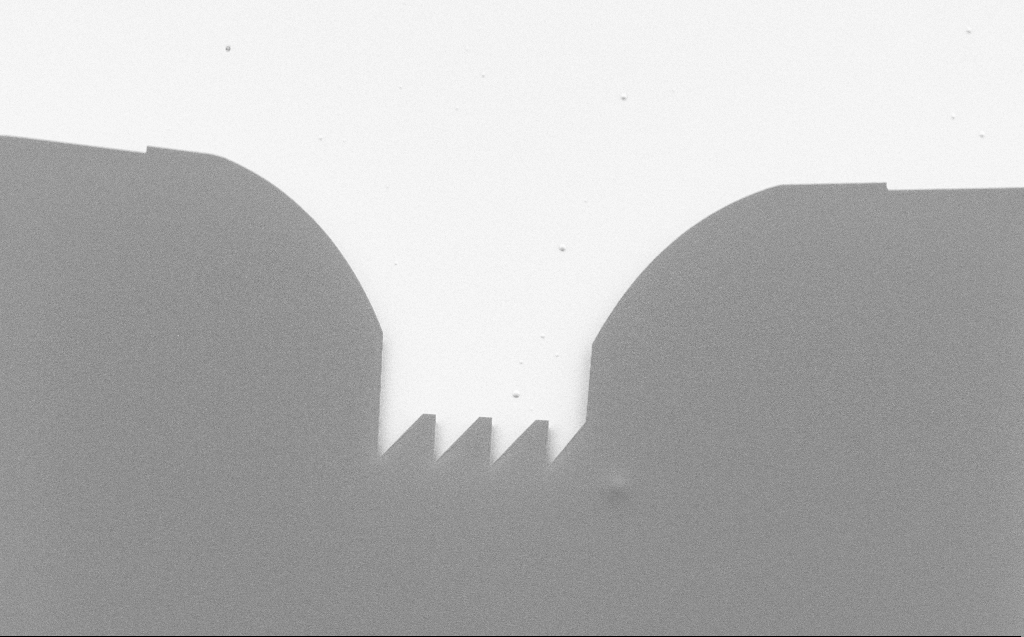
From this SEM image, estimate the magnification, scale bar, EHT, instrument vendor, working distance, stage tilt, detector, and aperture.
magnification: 1.82 K X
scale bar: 20000 nm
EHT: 1.1 kV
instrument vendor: Zeiss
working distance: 6 mm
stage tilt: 30°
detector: SE2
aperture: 30 µm